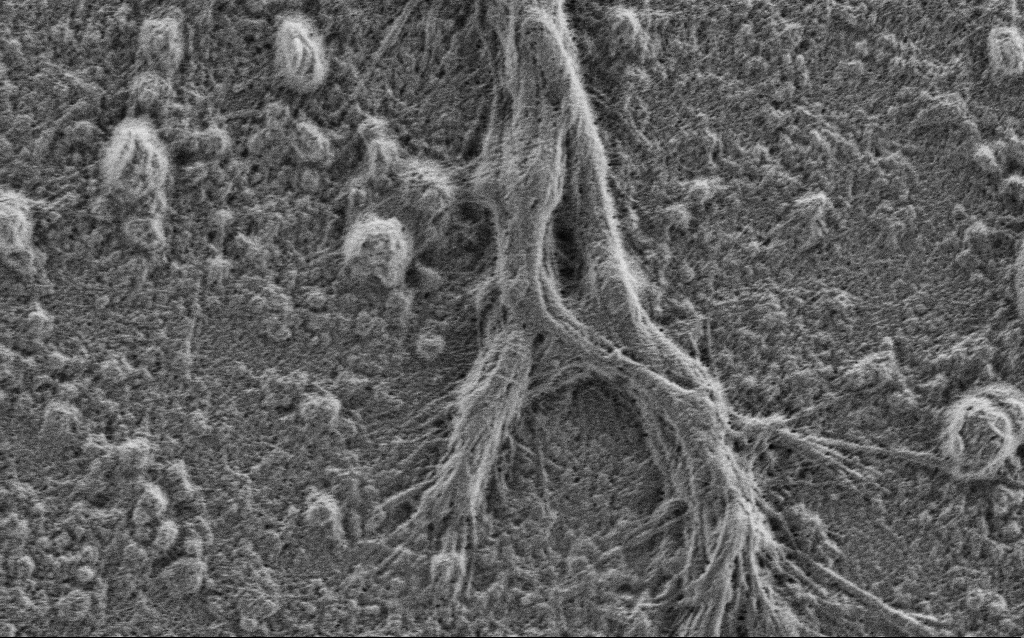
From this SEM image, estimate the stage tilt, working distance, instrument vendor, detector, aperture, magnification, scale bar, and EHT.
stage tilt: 0°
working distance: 4 mm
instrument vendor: Zeiss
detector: SE2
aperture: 30 µm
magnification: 10 K X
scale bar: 2000 nm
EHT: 0.9 kV